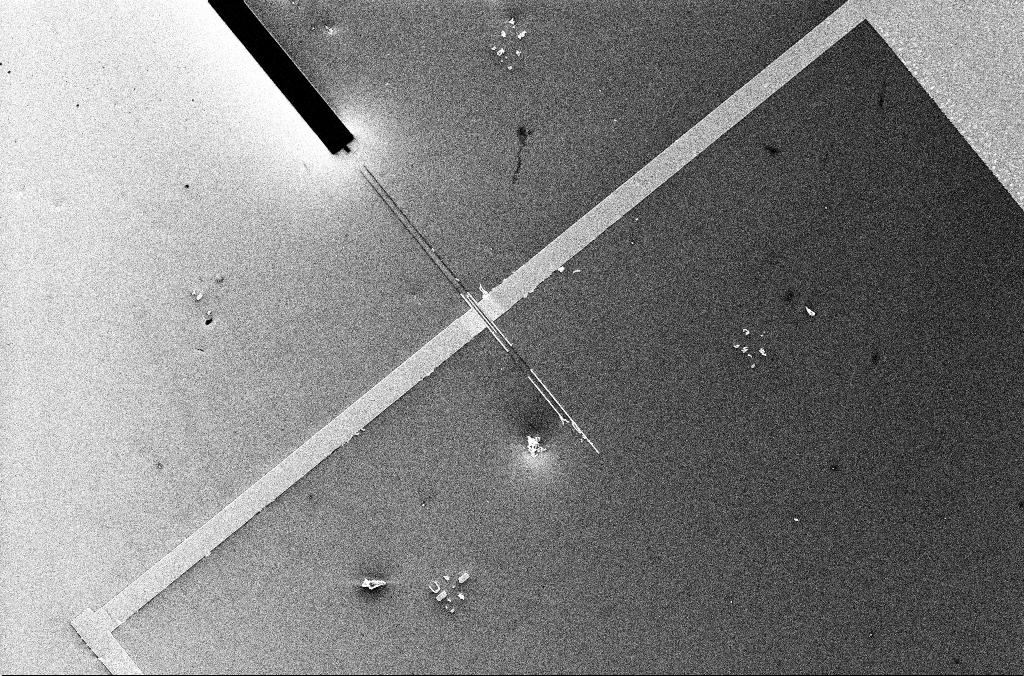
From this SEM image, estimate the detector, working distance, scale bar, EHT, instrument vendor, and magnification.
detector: InLens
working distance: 3.3 mm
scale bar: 20000 nm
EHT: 5 kV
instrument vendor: Zeiss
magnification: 1.79 K X